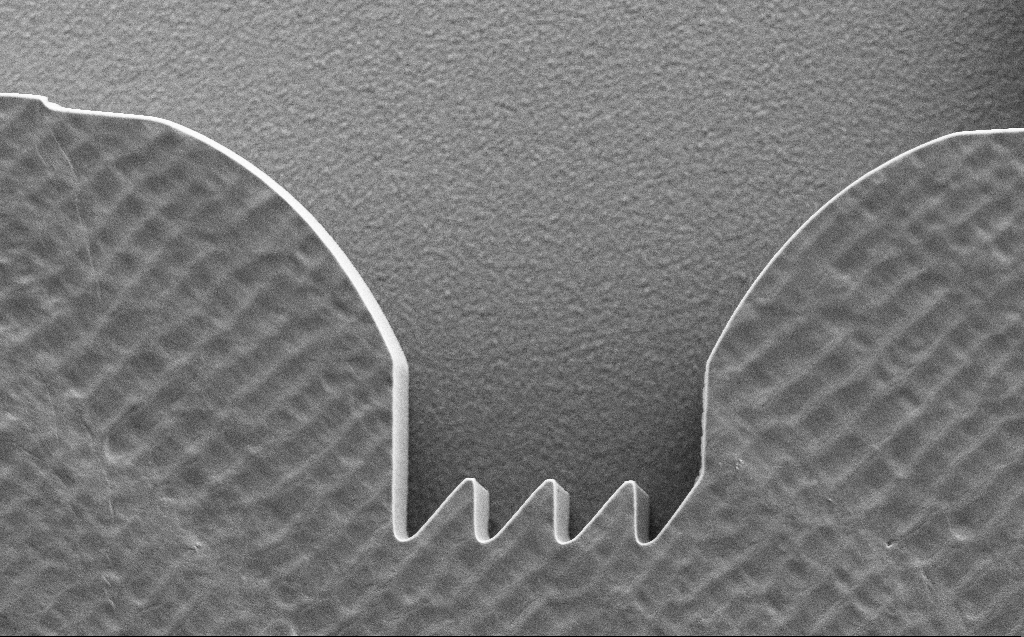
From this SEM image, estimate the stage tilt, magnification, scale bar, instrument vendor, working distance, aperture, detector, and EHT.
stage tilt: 0°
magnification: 2.56 K X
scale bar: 20000 nm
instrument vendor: Zeiss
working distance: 6 mm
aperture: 30 µm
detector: SE2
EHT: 1 kV